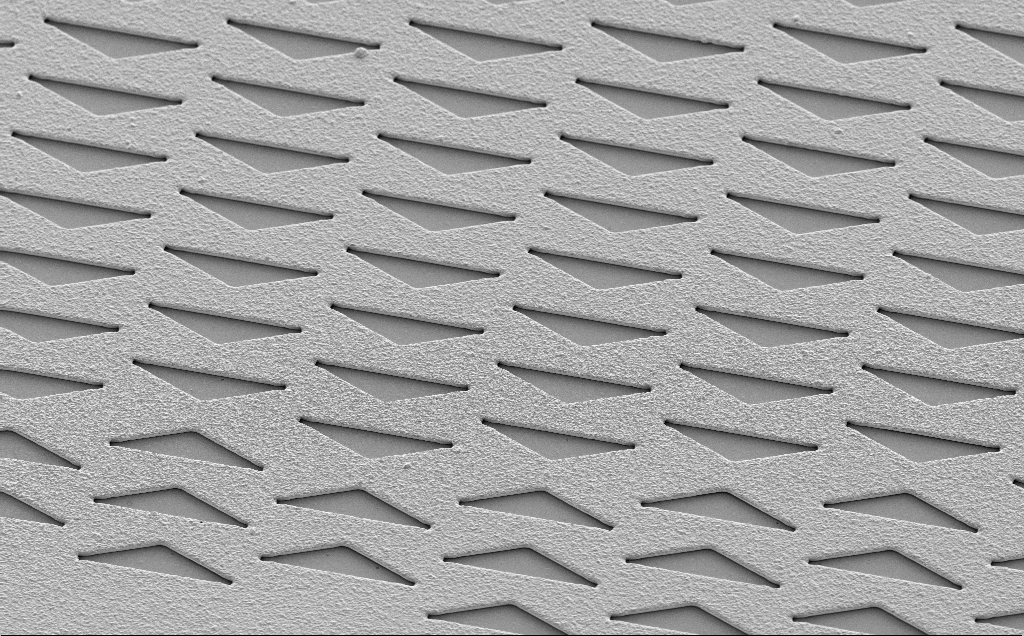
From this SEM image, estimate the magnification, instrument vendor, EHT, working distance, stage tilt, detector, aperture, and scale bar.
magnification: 1.54 K X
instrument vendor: Zeiss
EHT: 5 kV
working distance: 13 mm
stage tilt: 35°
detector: SE2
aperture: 30 µm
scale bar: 10000 nm